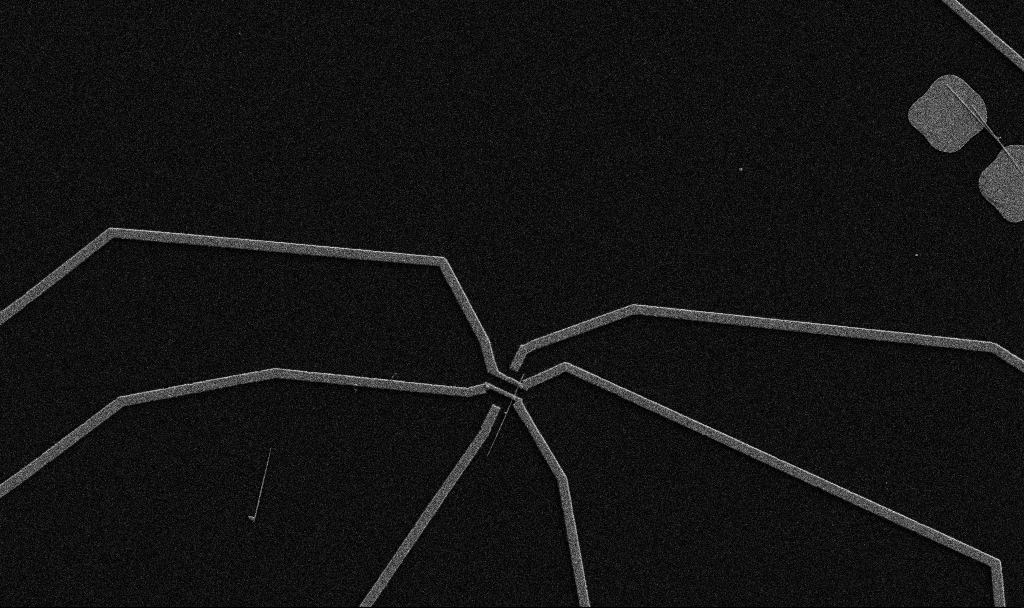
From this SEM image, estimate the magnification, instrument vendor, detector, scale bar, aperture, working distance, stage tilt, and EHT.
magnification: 5 K X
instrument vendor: Zeiss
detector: SE2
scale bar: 10000 nm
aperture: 30 µm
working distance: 10.7 mm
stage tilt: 0°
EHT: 5 kV